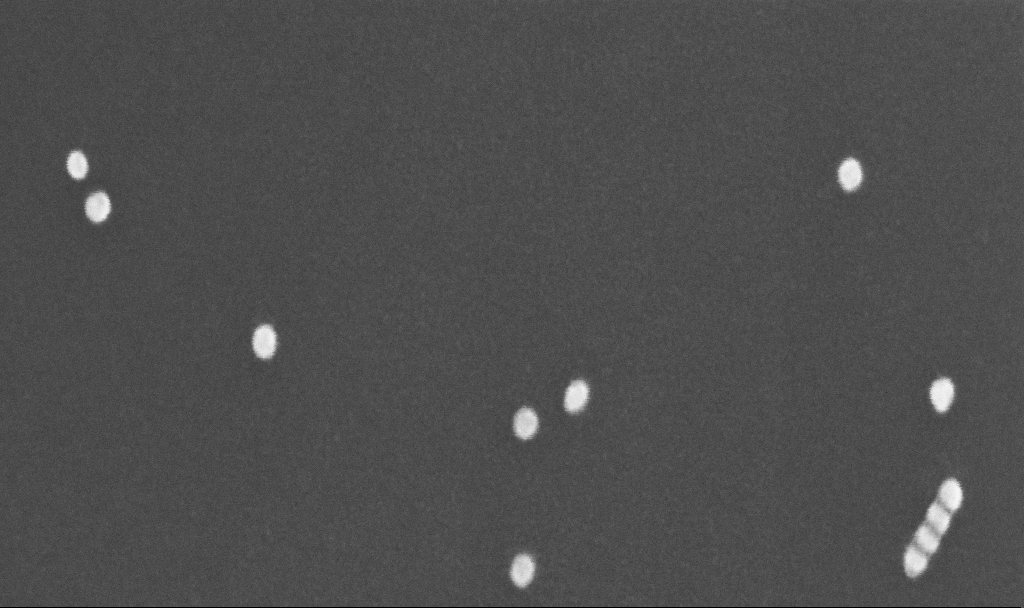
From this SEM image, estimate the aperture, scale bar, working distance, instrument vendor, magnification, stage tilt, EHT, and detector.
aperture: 30 µm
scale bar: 100 nm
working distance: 3.3 mm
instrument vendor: Zeiss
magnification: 394.49 K X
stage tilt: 0°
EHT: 10 kV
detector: InLens